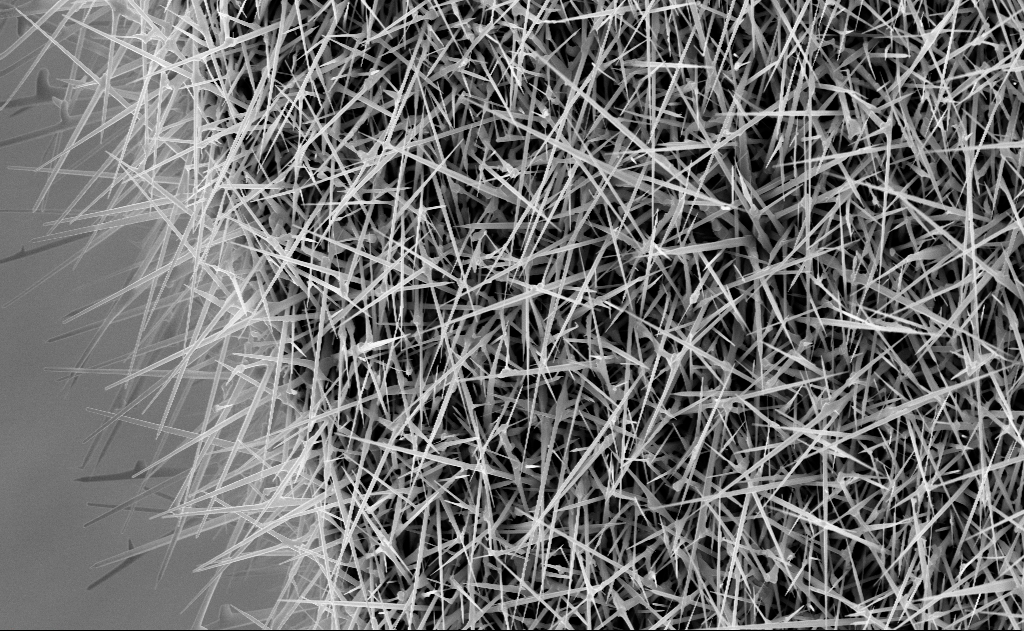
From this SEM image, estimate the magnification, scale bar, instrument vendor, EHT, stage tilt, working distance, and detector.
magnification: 10 K X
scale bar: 2000 nm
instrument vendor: Zeiss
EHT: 10 kV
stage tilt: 0°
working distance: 10 mm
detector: InLens